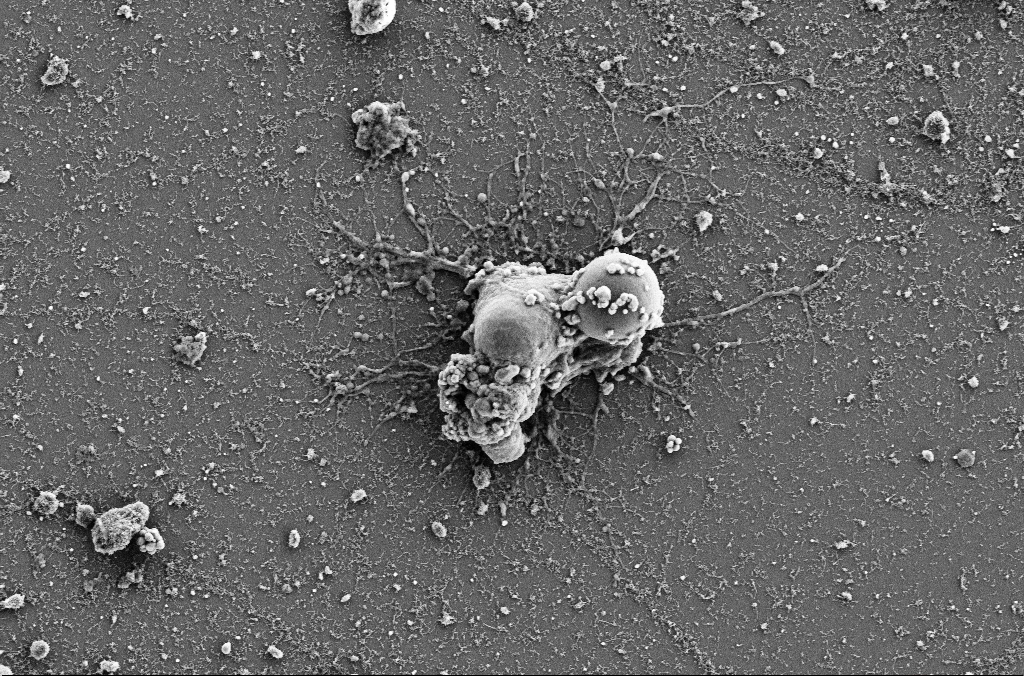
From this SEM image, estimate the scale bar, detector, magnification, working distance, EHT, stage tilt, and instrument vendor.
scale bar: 10000 nm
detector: SE2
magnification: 4 K X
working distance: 4 mm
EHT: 5 kV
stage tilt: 0°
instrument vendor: Zeiss